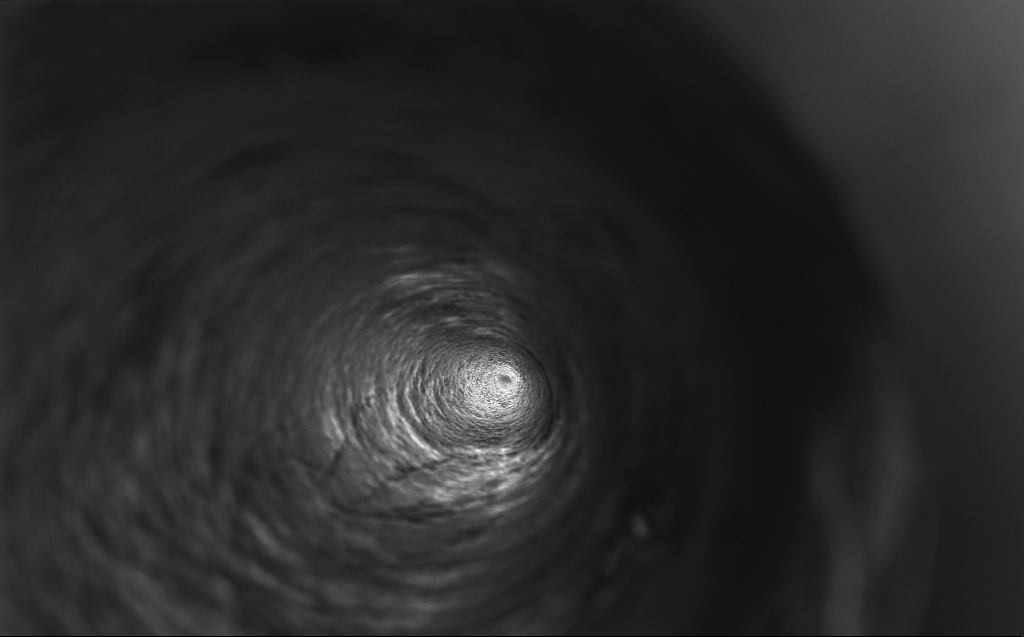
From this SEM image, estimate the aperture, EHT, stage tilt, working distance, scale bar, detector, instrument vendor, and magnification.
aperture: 30 µm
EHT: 10 kV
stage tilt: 0°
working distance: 4 mm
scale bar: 10000 nm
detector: InLens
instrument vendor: Zeiss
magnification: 2.54 K X